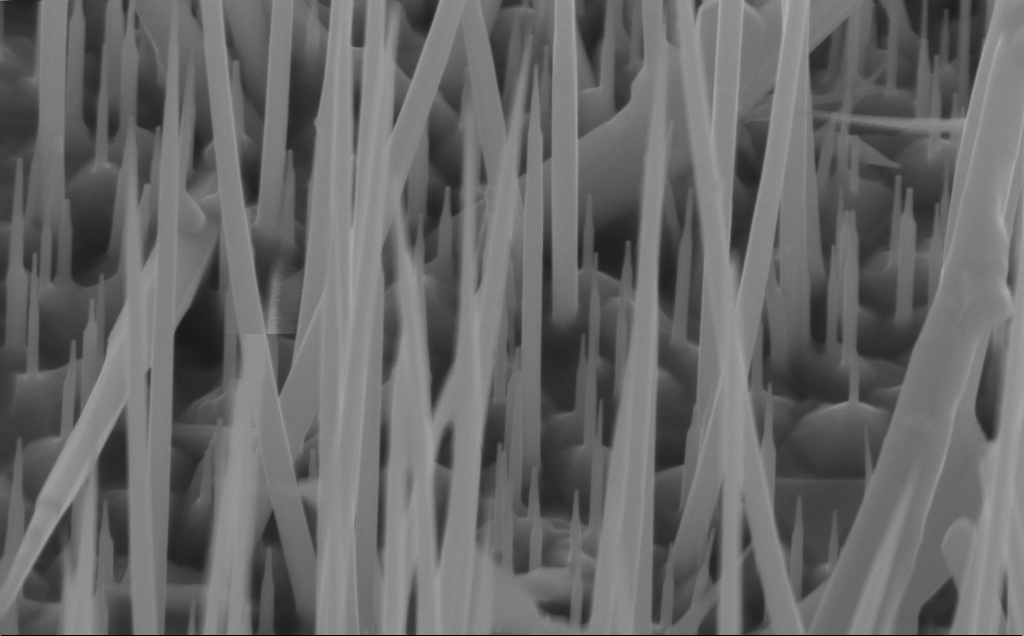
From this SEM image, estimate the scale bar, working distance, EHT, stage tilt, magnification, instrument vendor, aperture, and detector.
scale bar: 200 nm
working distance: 4 mm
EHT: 10 kV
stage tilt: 45°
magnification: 73.37 K X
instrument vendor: Zeiss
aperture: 30 µm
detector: InLens